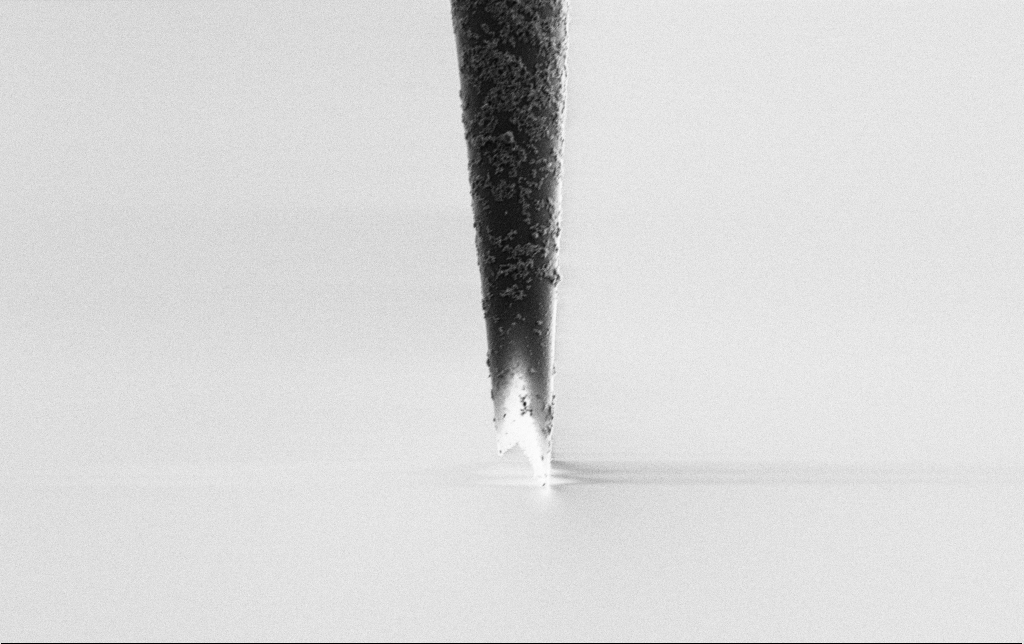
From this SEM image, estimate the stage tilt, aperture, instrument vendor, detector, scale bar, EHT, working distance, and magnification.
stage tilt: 0°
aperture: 30 µm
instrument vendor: Zeiss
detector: SE2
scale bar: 2000 nm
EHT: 2 kV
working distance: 6.5 mm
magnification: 10 K X